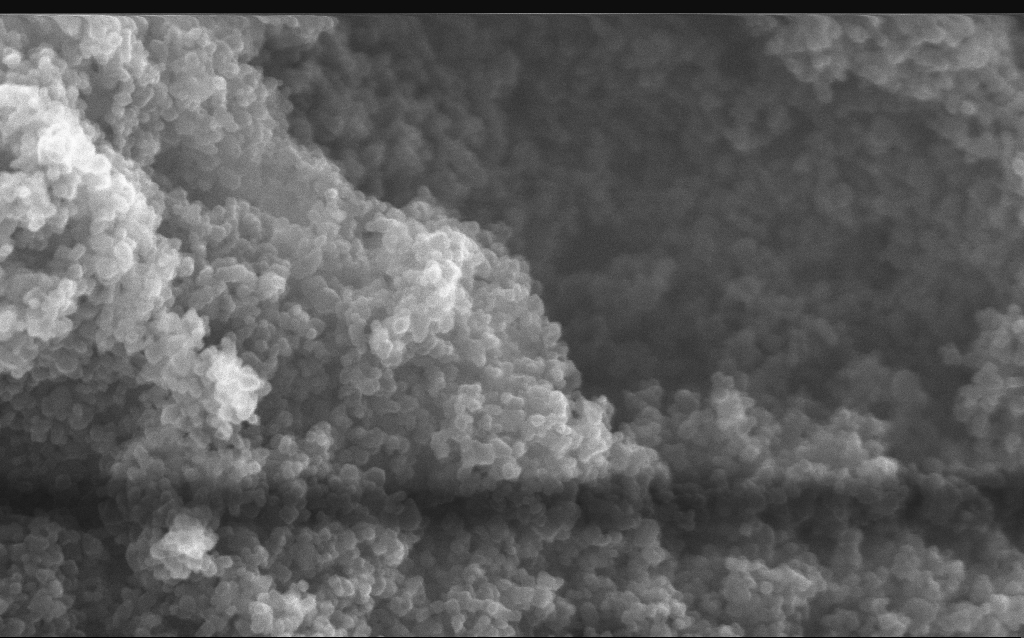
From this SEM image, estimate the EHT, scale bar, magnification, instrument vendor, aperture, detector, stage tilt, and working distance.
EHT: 10 kV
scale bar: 100 nm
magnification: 211.33 K X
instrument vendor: Zeiss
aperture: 30 µm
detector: InLens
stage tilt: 0°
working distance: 2.7 mm